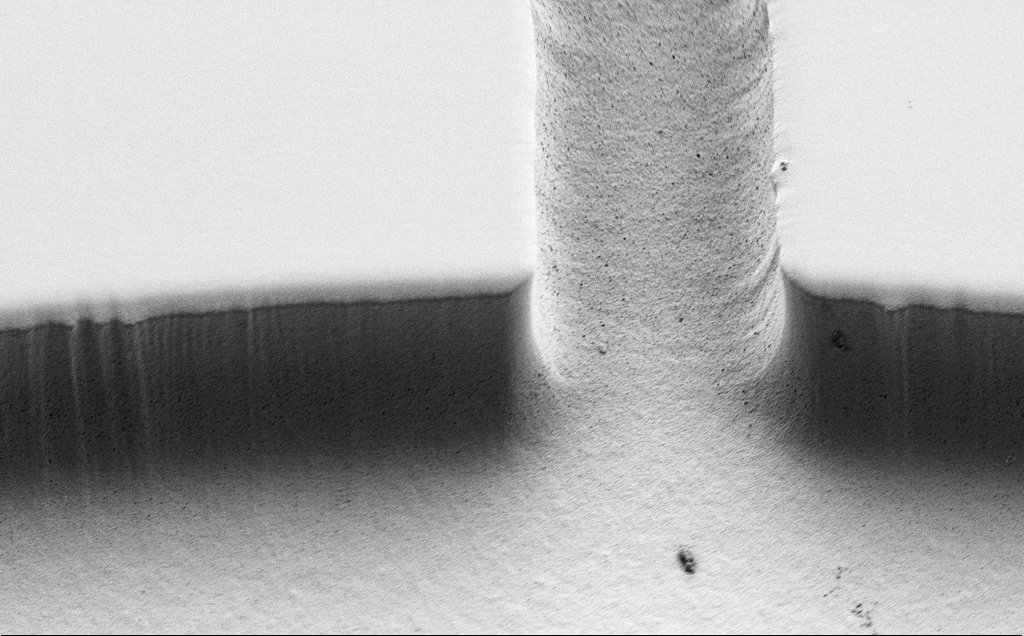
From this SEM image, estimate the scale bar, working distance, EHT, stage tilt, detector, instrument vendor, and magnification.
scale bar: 10000 nm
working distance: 6 mm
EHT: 0.8 kV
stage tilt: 45°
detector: SE2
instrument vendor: Zeiss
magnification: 2.02 K X